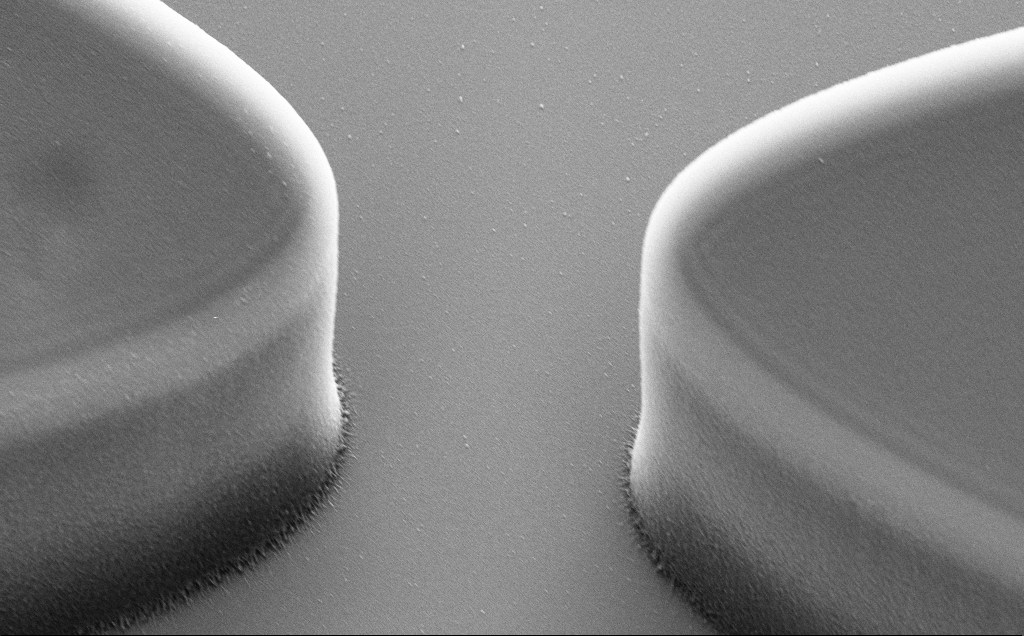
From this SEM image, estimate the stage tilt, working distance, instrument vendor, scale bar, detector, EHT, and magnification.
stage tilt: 35.3°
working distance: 11 mm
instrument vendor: Zeiss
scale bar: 2000 nm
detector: SE2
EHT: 10 kV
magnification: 9.54 K X